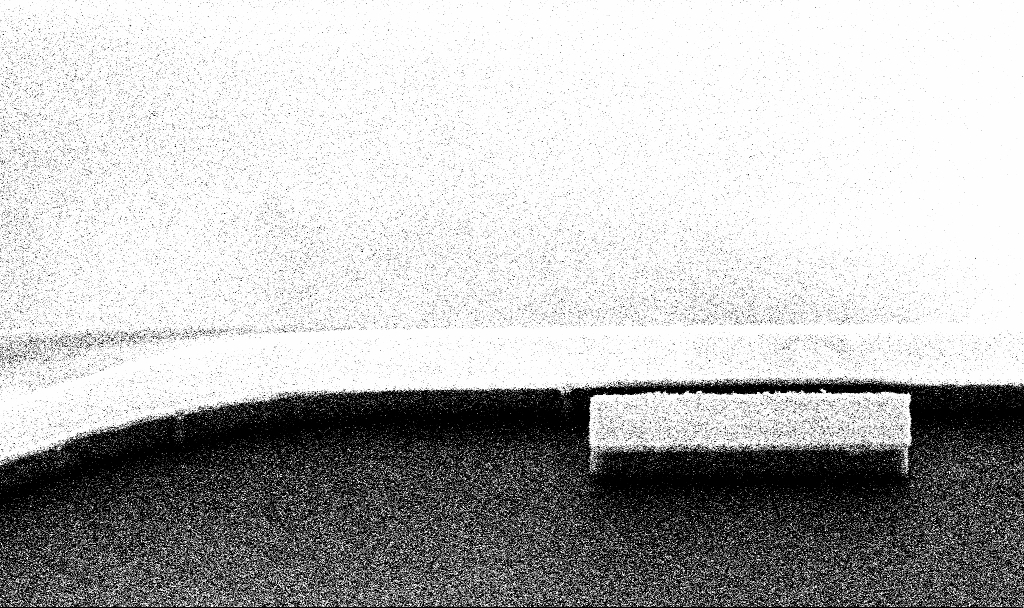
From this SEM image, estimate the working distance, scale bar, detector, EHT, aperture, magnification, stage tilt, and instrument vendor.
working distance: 7.1 mm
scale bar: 1000 nm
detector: SE2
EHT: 3 kV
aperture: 30 µm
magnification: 39.49 K X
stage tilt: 45°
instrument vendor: Zeiss